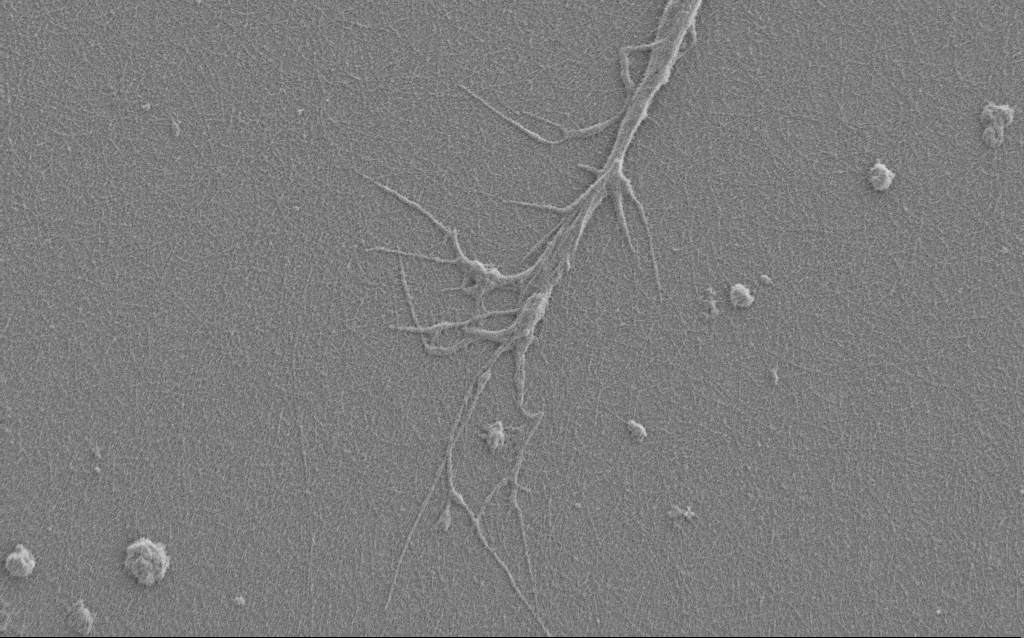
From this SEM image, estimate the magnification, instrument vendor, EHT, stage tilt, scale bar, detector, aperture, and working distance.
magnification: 7.5 K X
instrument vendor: Zeiss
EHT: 1 kV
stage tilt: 0°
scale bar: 2000 nm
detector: SE2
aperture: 30 µm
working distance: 6 mm